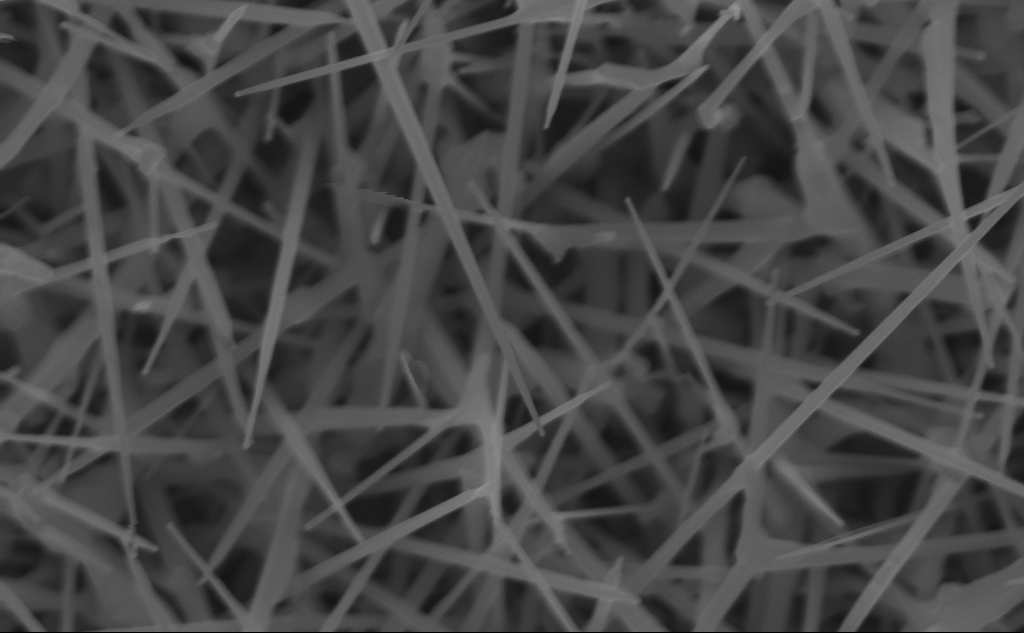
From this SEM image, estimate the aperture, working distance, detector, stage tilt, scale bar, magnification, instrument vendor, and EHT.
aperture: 30 µm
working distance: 7 mm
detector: InLens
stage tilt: -0°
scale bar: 200 nm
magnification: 80.28 K X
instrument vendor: Zeiss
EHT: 10 kV